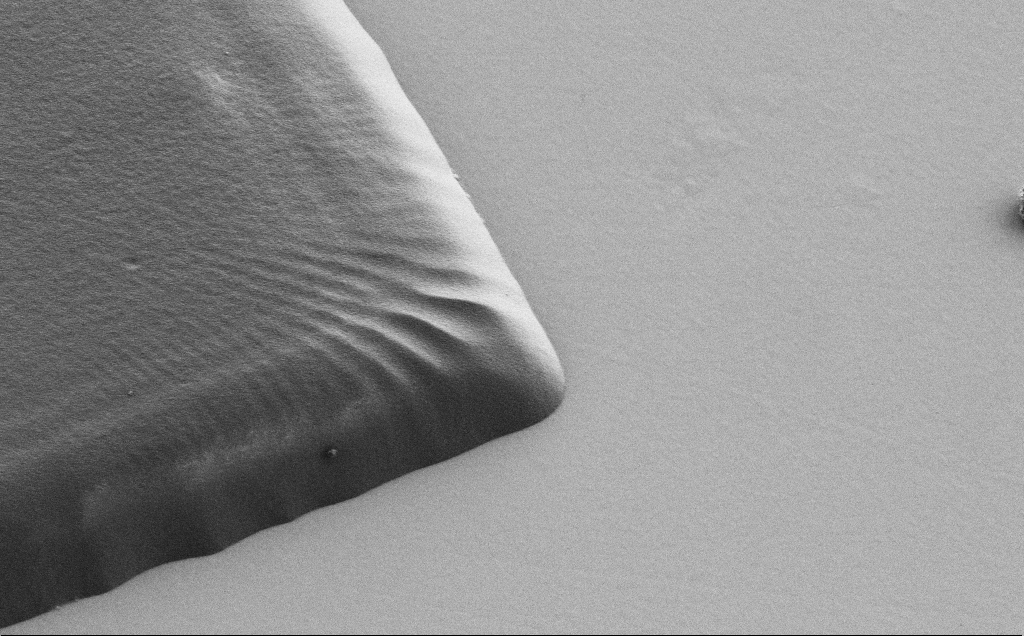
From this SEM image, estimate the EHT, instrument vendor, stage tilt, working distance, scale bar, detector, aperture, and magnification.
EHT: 1.2 kV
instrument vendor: Zeiss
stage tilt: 30°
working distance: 6 mm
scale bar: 10000 nm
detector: SE2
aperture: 30 µm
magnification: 5.67 K X